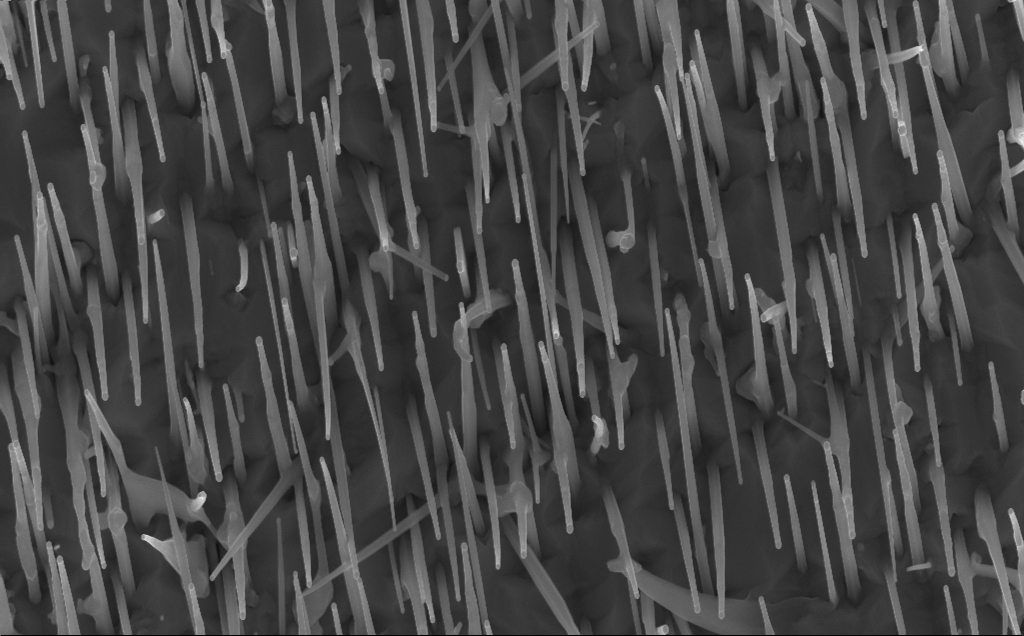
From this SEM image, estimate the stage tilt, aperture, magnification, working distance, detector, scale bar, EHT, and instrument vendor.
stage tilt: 0°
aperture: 30 µm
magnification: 40 K X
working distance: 7 mm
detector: InLens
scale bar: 1000 nm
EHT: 10 kV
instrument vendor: Zeiss